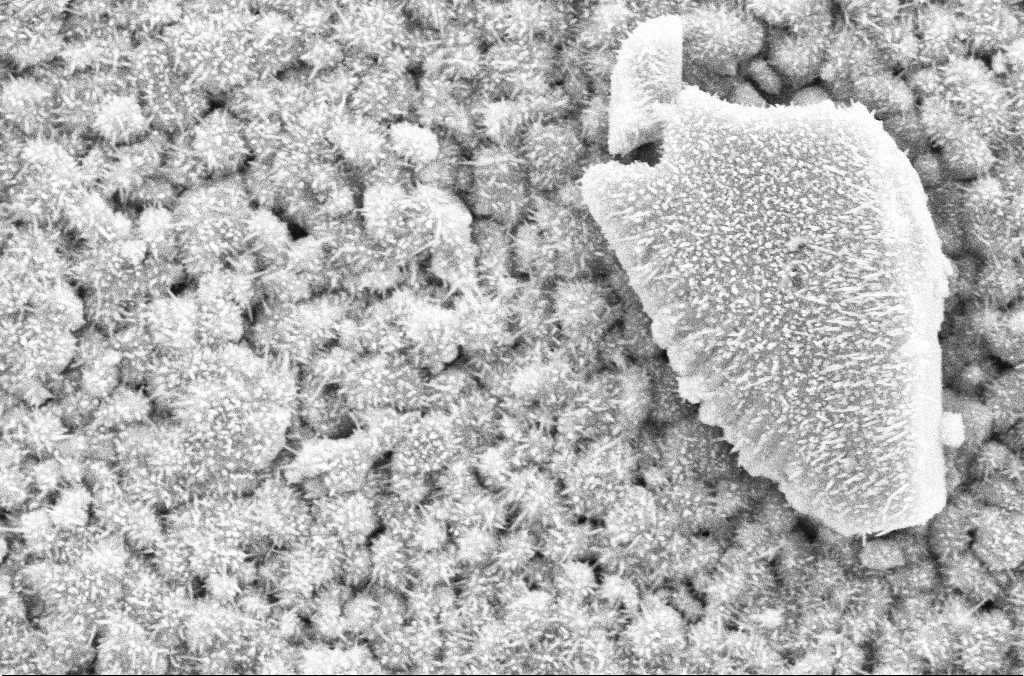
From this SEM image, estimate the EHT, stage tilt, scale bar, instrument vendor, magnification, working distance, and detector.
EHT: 10 kV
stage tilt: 0.1°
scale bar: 2000 nm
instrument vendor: Zeiss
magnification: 23.1 K X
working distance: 5.2 mm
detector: SE2